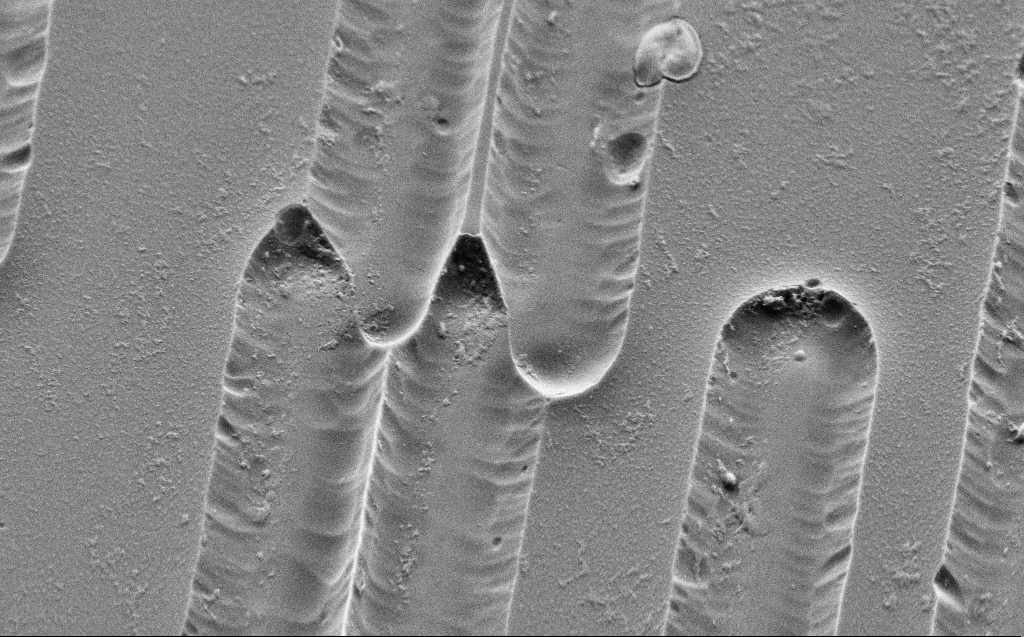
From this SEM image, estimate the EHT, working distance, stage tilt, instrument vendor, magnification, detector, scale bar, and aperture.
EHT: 3 kV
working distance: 5 mm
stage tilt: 45°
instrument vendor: Zeiss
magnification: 13.01 K X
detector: SE2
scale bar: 2000 nm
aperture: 30 µm